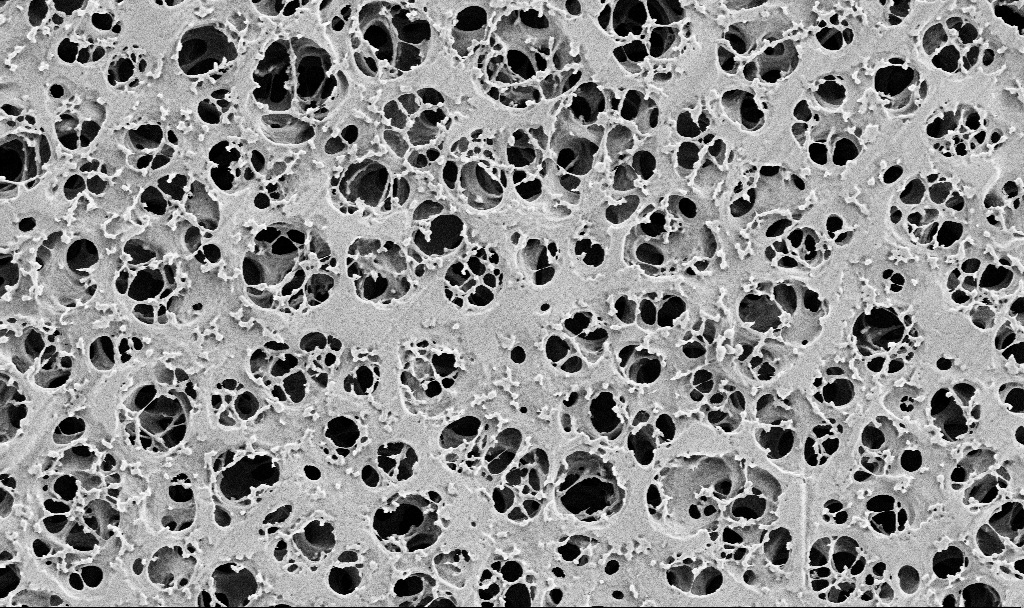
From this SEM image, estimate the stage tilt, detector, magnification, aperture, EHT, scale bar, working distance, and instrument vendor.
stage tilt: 0°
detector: SE2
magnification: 10 K X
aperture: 30 µm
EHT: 2 kV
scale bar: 2000 nm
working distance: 3.7 mm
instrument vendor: Zeiss